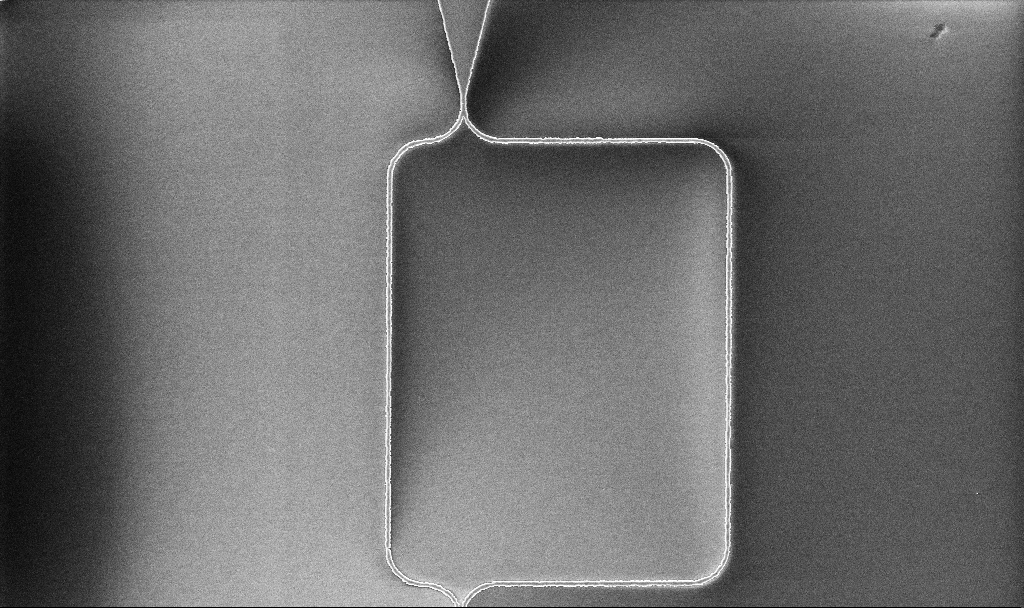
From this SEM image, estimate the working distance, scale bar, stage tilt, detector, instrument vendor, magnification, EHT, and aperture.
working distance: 10.1 mm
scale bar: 10000 nm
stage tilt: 0°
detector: InLens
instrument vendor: Zeiss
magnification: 2.86 K X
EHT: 5 kV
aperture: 30 µm